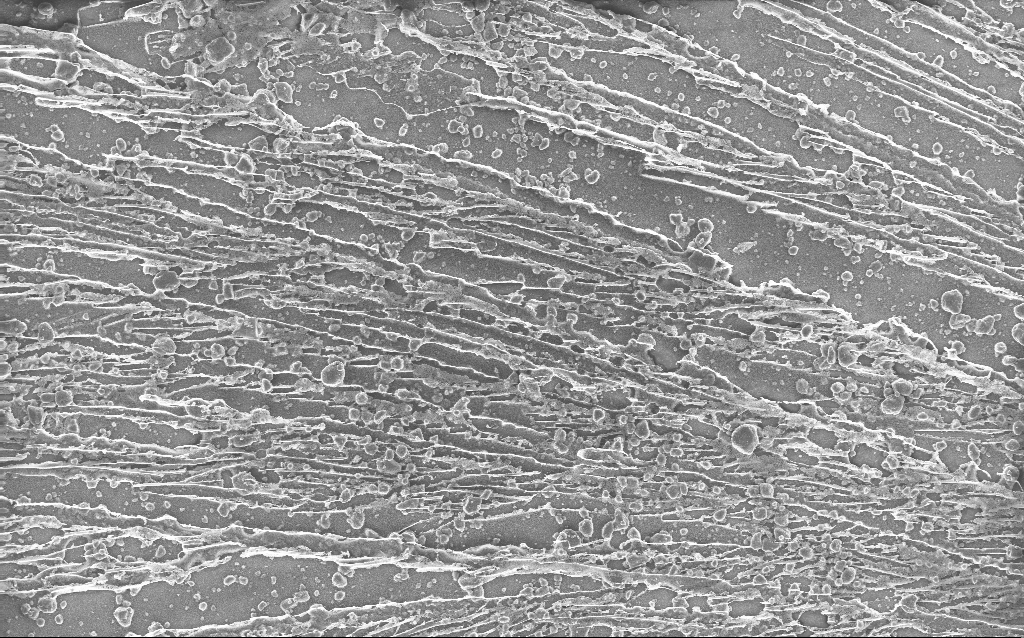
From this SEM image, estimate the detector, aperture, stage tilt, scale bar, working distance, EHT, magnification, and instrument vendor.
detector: InLens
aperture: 30 µm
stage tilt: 0°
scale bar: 20000 nm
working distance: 2.5 mm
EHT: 10 kV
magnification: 0.792 K X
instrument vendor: Zeiss